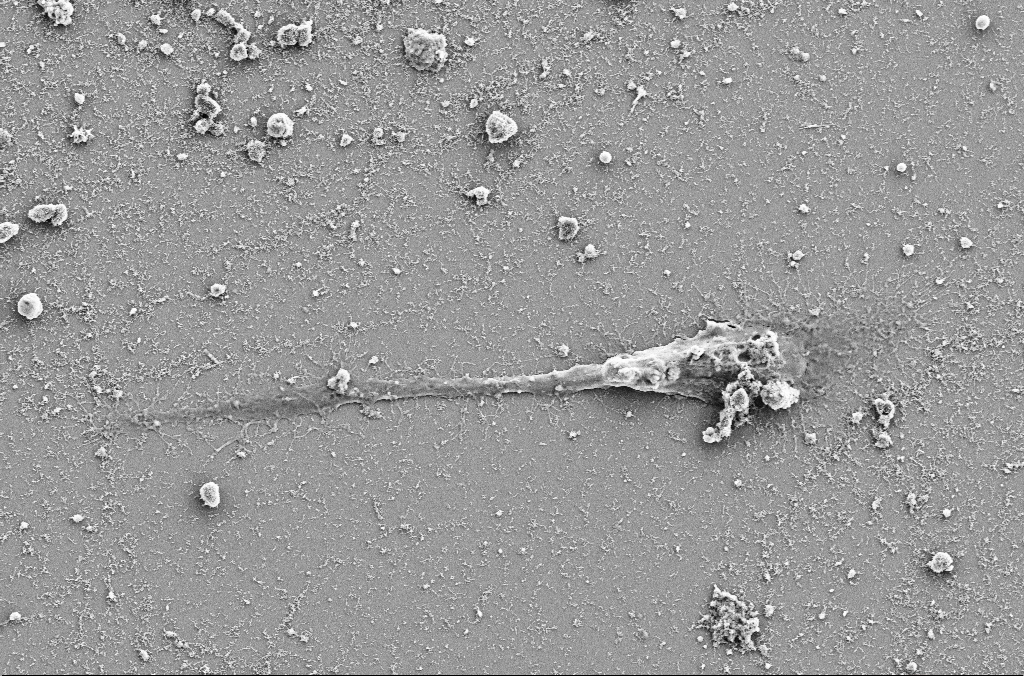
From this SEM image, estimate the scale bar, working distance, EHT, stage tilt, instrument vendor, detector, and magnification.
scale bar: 10000 nm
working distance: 4 mm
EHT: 5 kV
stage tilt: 0°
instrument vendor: Zeiss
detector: SE2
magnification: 3.5 K X